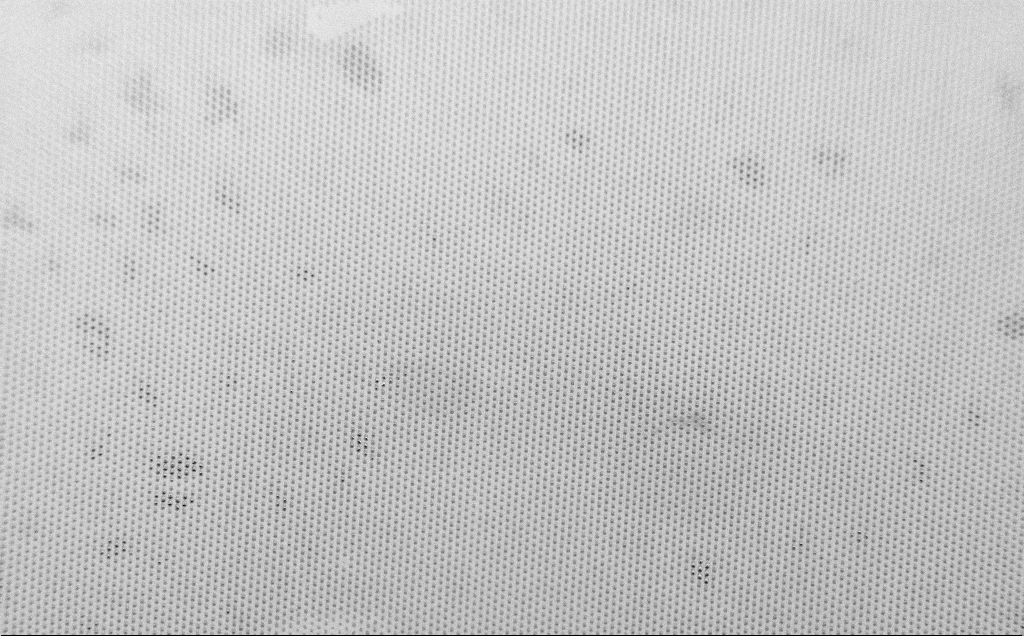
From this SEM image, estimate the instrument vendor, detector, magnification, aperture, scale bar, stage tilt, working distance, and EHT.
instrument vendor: Zeiss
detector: SE2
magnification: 0.295 K X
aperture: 30 µm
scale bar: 100000 nm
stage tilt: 45°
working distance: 7 mm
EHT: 1.5 kV